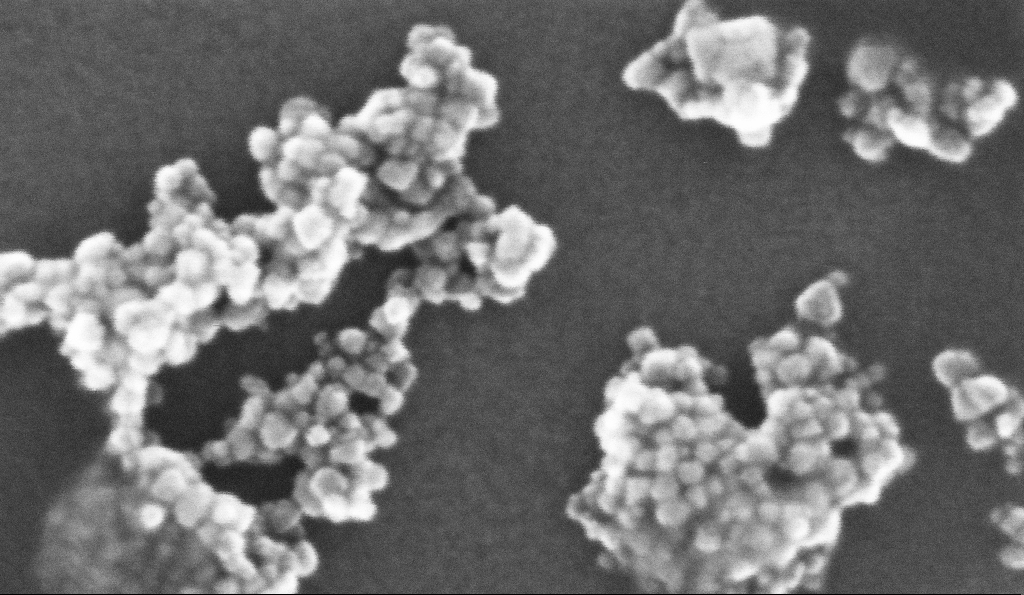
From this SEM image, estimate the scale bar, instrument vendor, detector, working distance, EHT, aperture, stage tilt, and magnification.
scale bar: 100 nm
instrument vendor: Zeiss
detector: InLens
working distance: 5.2 mm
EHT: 10 kV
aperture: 30 µm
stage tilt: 0°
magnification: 678.43 K X